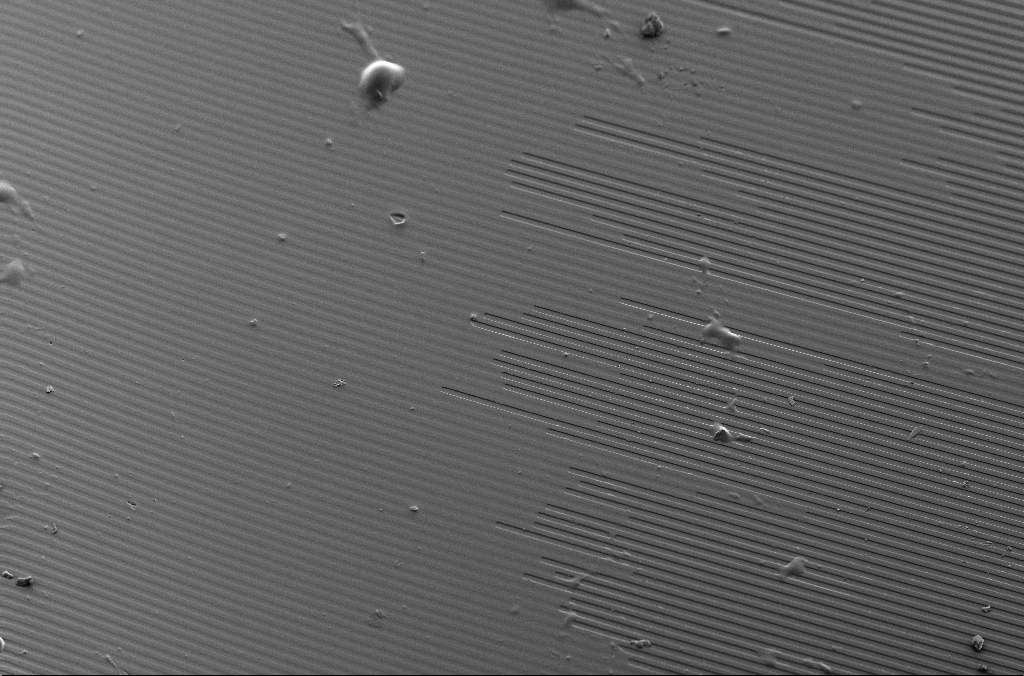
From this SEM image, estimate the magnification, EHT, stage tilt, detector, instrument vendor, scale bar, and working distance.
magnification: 0.2 K X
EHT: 5 kV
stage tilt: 40°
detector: SE2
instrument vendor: Zeiss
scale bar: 100000 nm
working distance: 7.2 mm